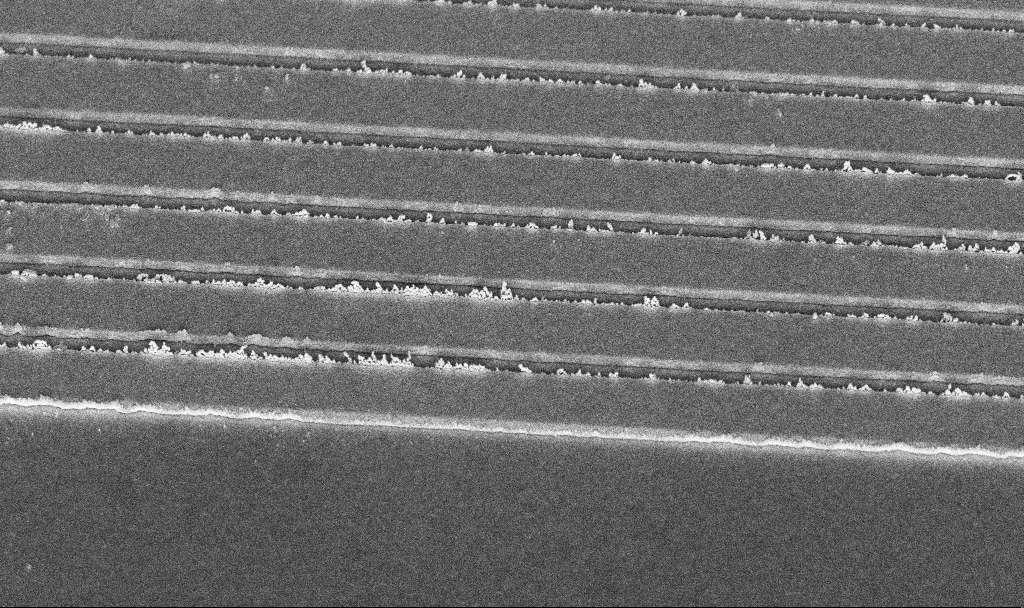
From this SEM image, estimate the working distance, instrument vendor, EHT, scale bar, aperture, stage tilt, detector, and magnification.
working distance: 7.5 mm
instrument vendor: Zeiss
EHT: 5 kV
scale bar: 2000 nm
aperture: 30 µm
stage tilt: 45°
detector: InLens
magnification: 34.38 K X